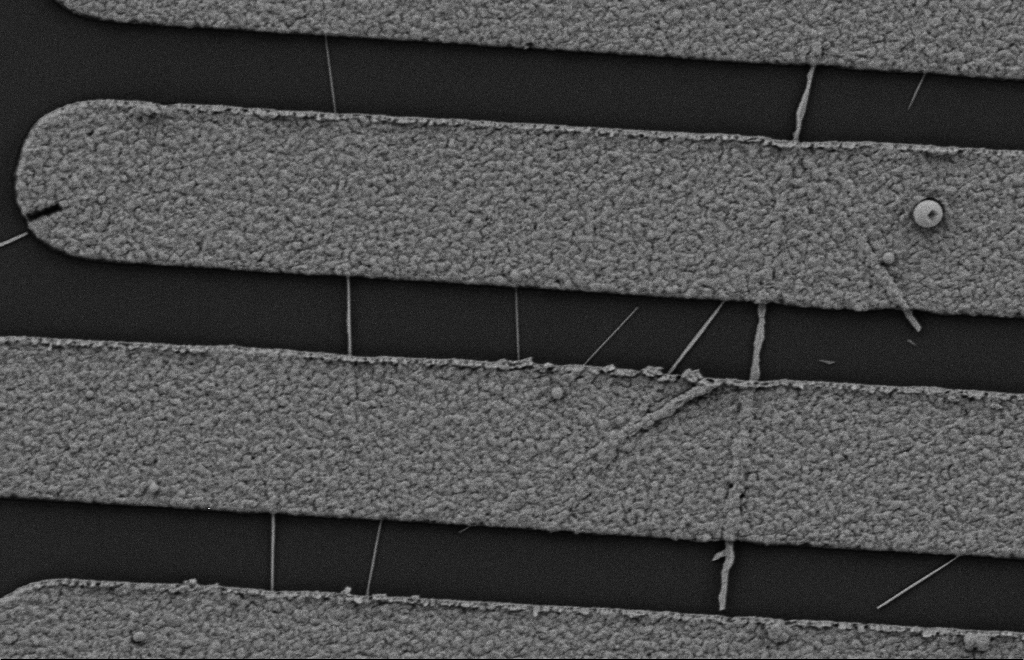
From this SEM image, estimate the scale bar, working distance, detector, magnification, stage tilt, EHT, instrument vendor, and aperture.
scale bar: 2000 nm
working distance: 10 mm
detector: SE2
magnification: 21.93 K X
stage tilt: -0.3°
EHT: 2 kV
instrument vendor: Zeiss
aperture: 20 µm